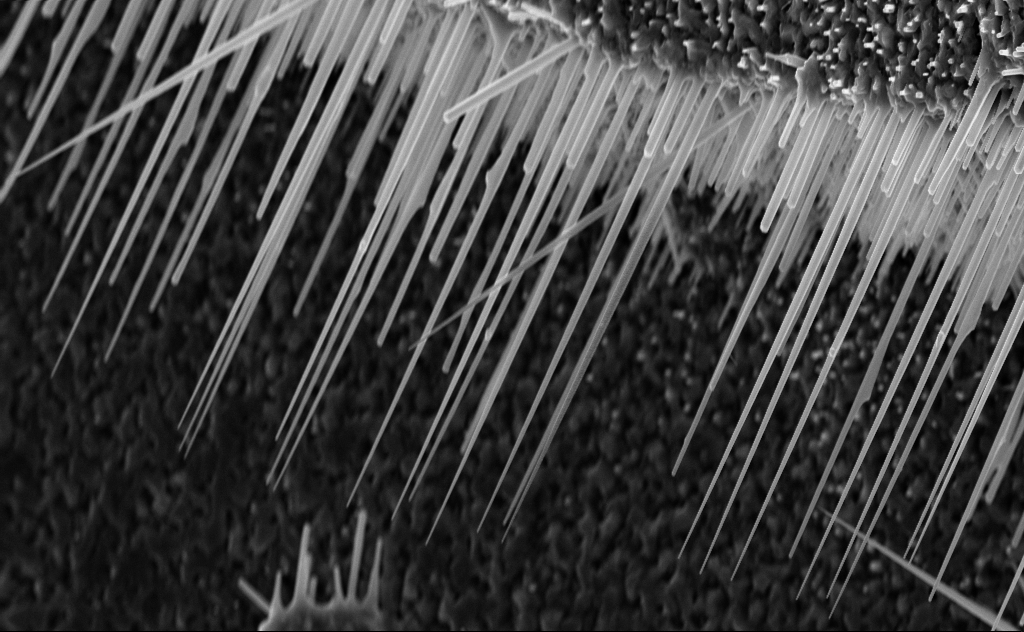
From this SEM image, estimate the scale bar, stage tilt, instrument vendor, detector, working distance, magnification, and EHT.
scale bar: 2000 nm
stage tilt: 0°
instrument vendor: Zeiss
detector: InLens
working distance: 8 mm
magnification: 15 K X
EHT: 10 kV